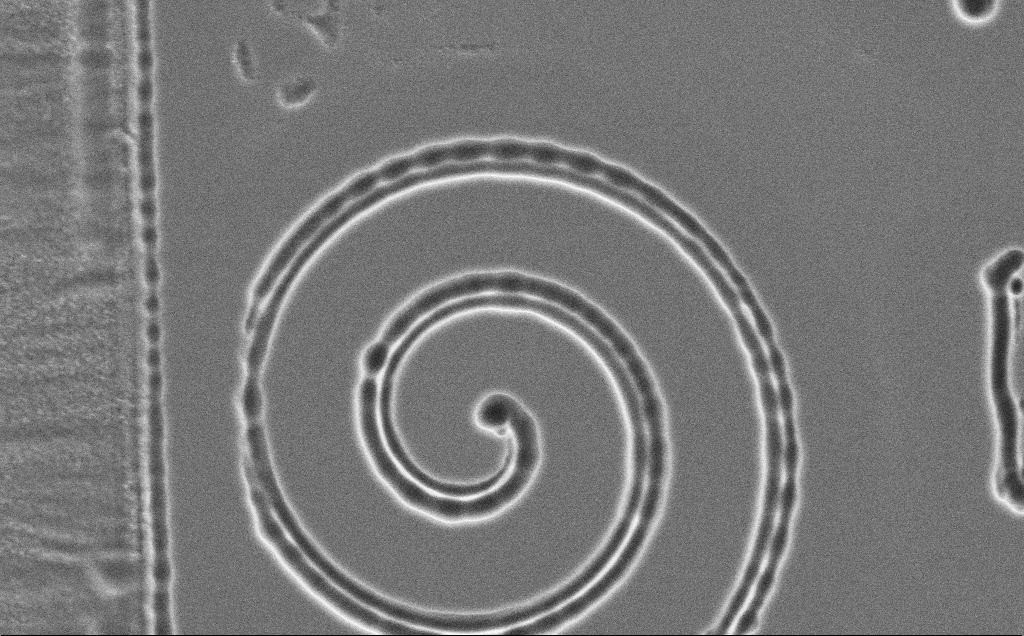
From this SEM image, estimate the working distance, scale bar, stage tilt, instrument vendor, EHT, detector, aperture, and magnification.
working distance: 13 mm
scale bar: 2000 nm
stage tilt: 0°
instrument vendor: Zeiss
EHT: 5 kV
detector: SE2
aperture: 30 µm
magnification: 7.86 K X